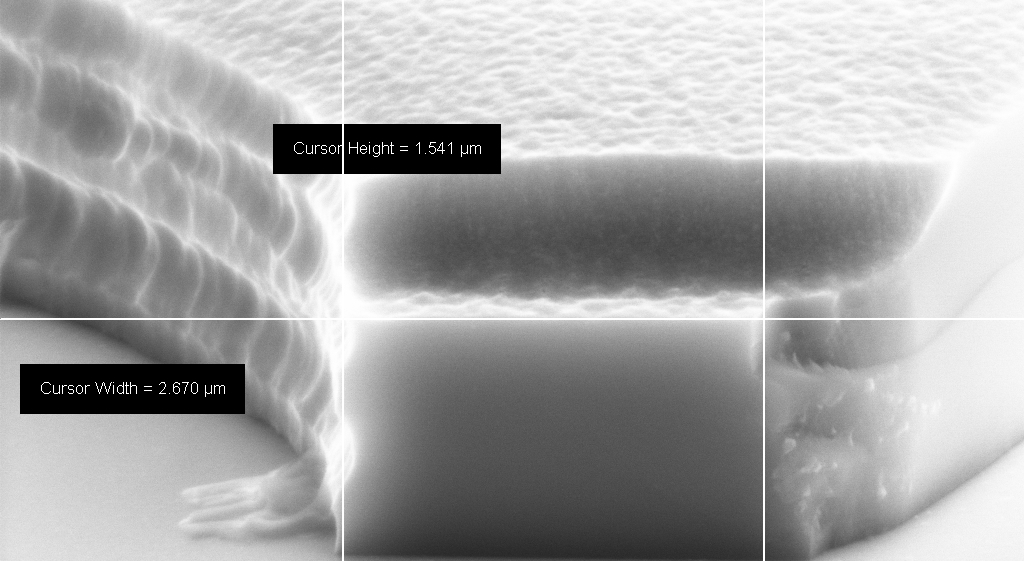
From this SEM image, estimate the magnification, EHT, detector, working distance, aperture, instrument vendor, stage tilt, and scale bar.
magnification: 57.89 K X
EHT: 8 kV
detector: SE2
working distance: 12 mm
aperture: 30 µm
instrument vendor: Zeiss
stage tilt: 70°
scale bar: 1000 nm